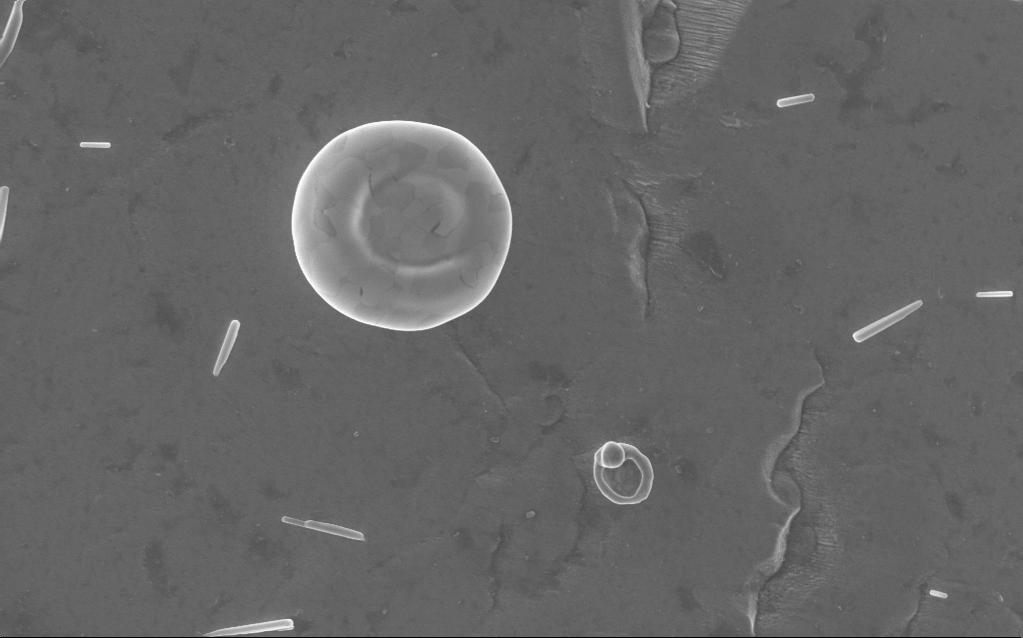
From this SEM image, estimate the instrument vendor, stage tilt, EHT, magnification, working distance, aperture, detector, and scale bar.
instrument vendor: Zeiss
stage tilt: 0°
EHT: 5 kV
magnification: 24 K X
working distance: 3 mm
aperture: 30 µm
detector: InLens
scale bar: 2000 nm